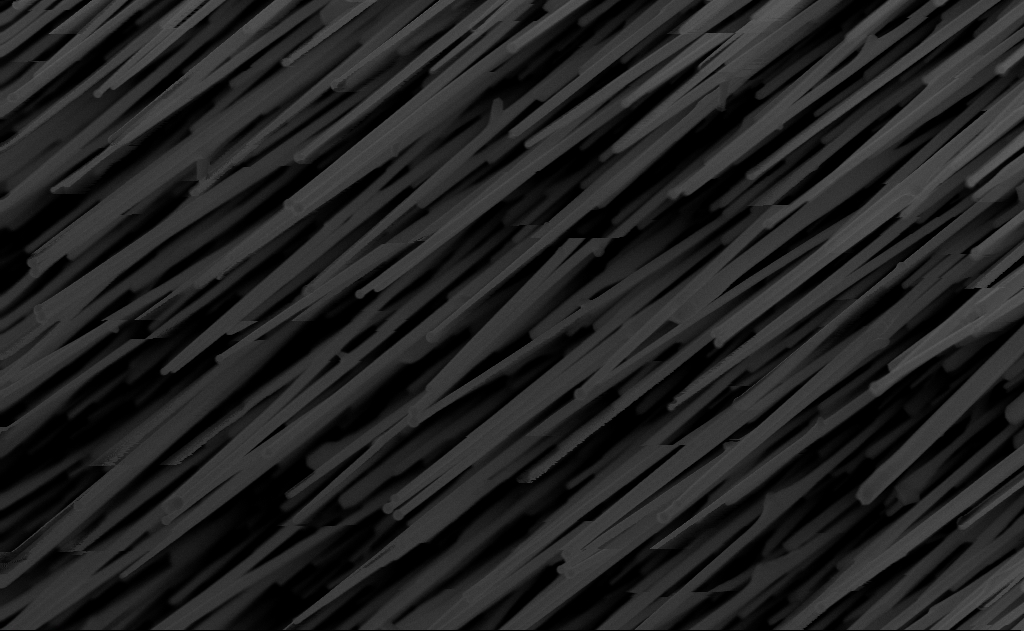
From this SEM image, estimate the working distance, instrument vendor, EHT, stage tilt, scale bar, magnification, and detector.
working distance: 10 mm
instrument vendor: Zeiss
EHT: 10 kV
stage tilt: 0°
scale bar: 1000 nm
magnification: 40 K X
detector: InLens